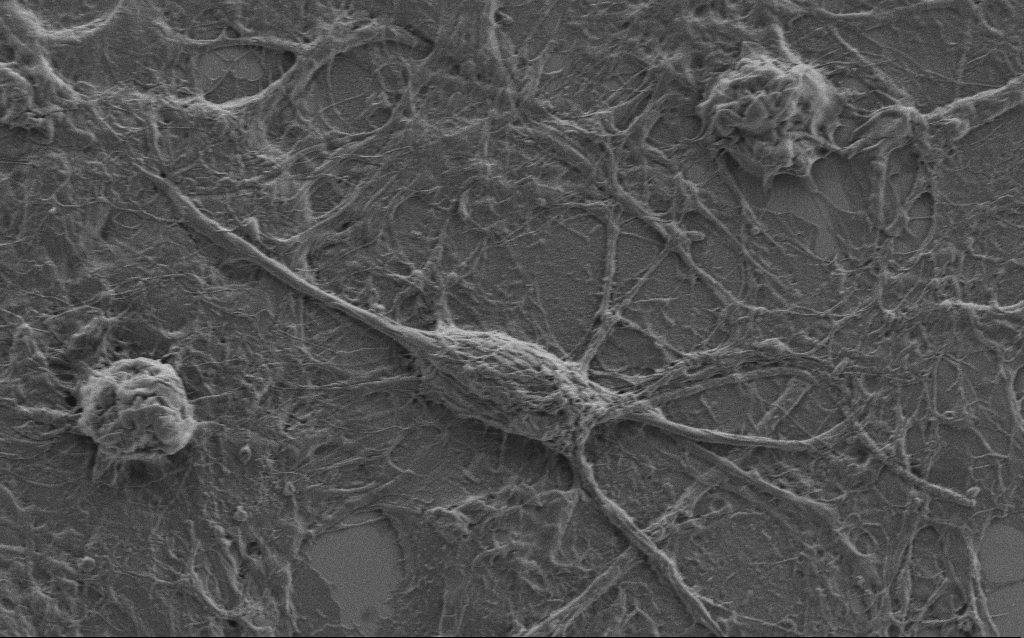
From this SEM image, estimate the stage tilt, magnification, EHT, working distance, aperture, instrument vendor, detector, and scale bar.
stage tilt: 0°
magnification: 5 K X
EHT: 1.5 kV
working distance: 6 mm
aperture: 30 µm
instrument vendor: Zeiss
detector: SE2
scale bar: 10000 nm